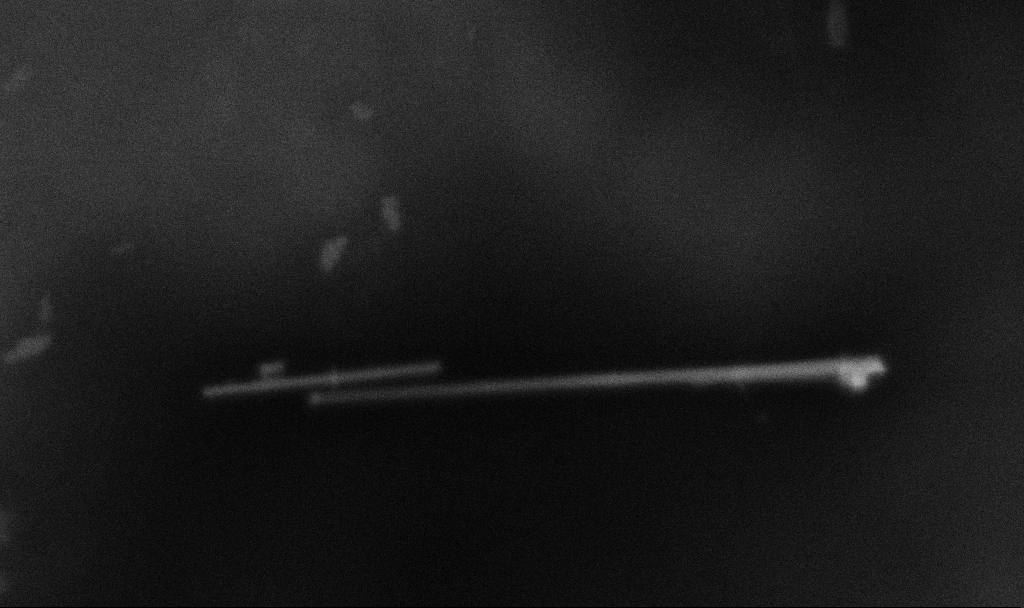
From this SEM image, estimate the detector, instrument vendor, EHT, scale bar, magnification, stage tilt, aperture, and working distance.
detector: InLens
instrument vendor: Zeiss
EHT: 3 kV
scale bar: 200 nm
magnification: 154.88 K X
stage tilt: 0°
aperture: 30 µm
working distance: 3.3 mm